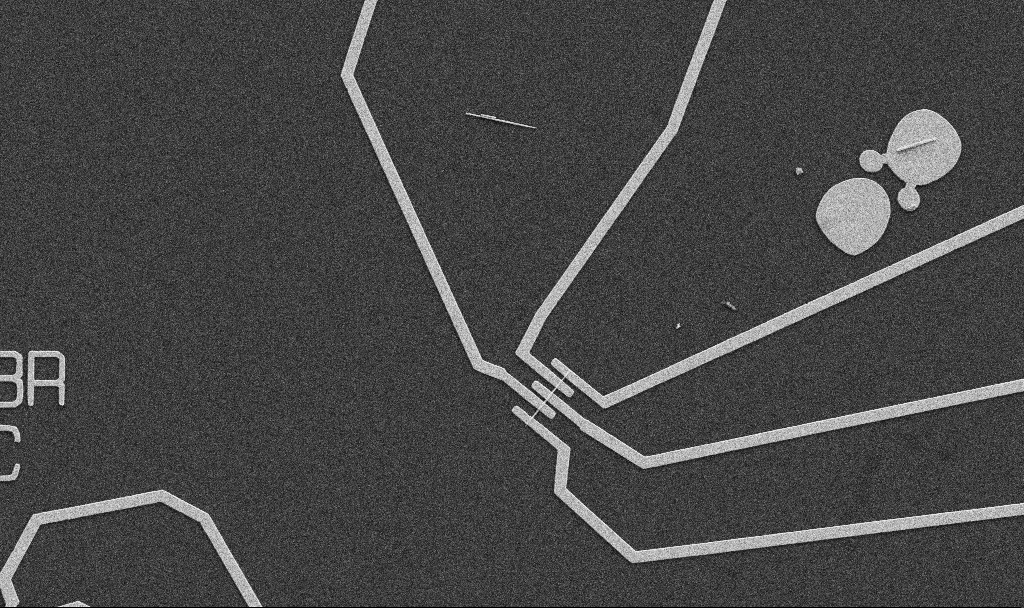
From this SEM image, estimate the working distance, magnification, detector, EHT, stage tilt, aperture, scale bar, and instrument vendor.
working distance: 10.7 mm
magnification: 5 K X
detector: SE2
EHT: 5 kV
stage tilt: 0°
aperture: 30 µm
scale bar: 10000 nm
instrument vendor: Zeiss